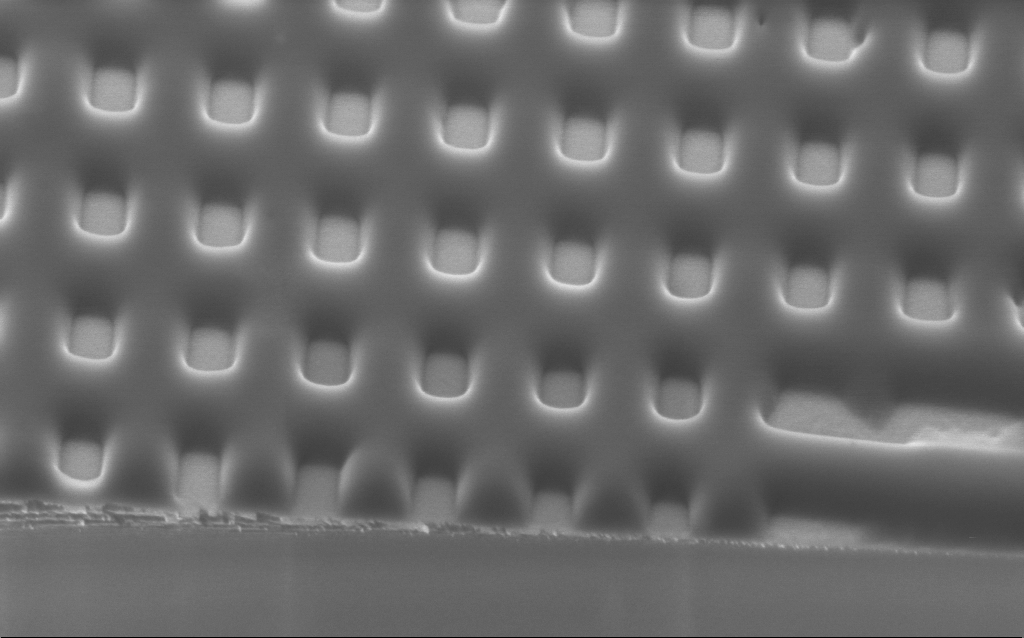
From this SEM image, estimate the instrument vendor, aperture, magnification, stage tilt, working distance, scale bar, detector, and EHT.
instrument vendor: Zeiss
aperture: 30 µm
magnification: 87.38 K X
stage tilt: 45°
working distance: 2.7 mm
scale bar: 200 nm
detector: InLens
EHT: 2 kV